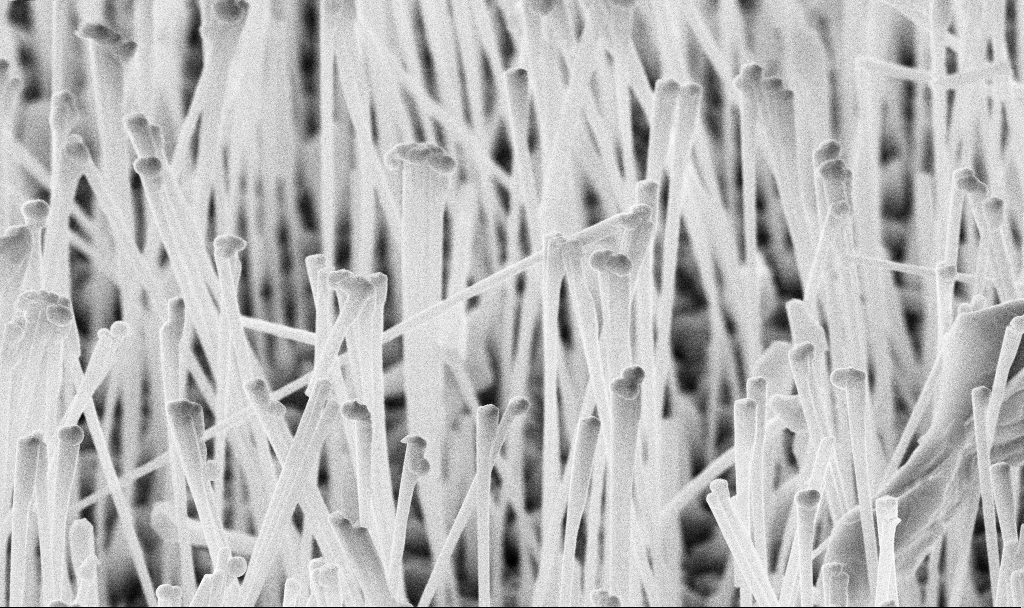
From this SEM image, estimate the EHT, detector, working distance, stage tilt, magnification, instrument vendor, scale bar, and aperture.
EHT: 10 kV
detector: InLens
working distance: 7 mm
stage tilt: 45°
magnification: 37.89 K X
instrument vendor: Zeiss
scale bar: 1000 nm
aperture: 30 µm